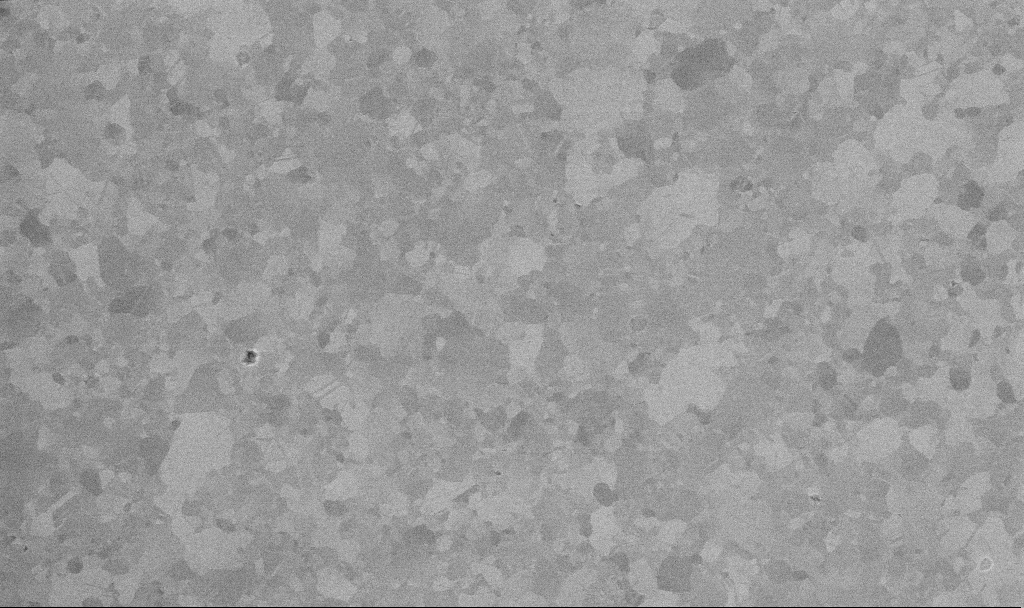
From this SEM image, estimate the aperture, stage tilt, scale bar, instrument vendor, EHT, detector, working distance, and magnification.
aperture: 30 µm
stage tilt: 0°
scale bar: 1000 nm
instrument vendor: Zeiss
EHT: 10 kV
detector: InLens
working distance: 3.4 mm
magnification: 50 K X